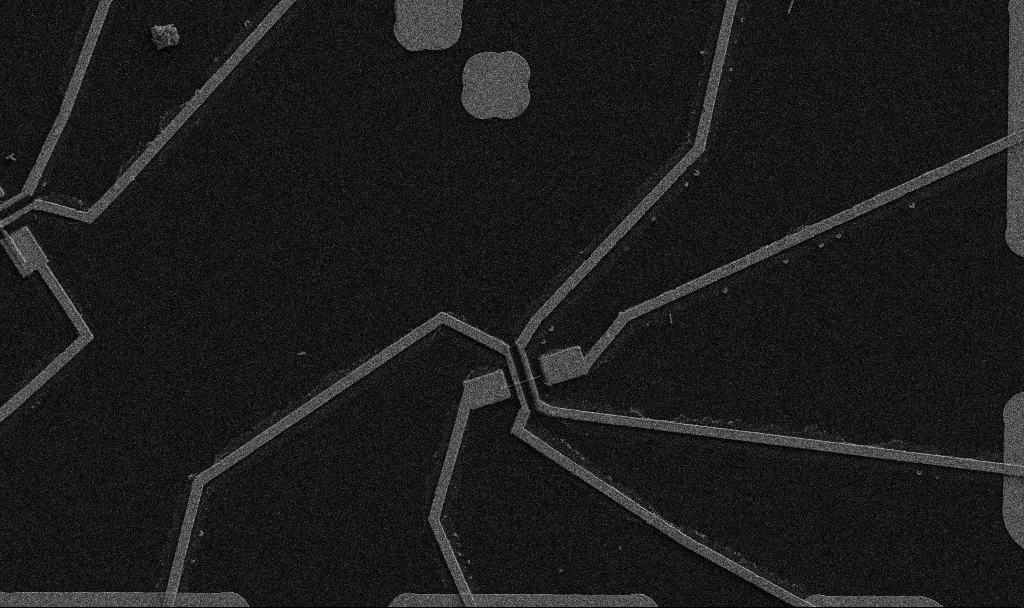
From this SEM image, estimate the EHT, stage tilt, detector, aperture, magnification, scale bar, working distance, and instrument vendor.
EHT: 5 kV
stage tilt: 0°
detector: SE2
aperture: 30 µm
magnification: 5 K X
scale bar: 10000 nm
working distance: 10.7 mm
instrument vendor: Zeiss